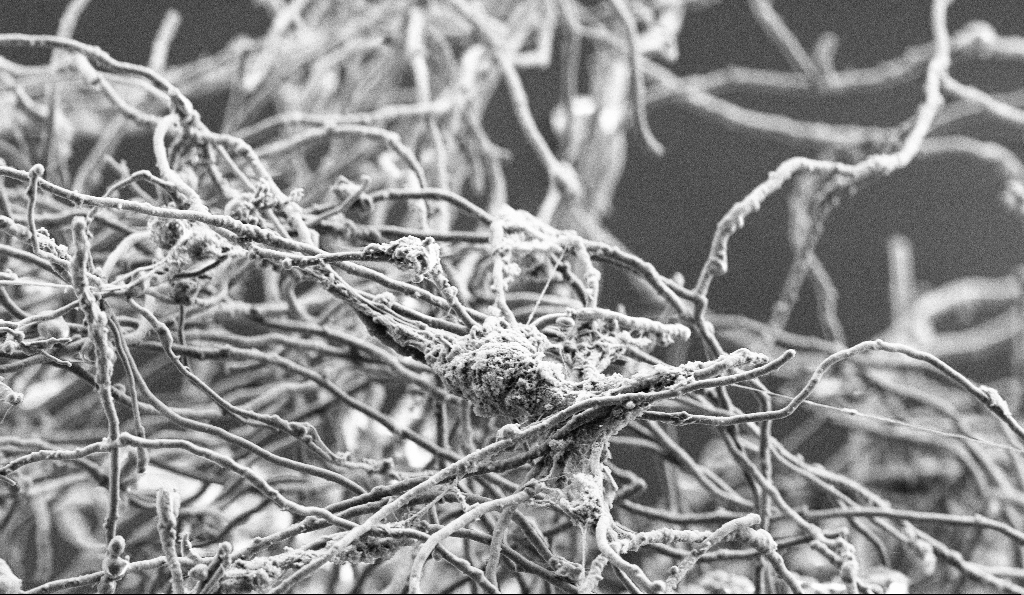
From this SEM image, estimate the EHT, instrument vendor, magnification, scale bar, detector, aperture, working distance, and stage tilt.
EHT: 3 kV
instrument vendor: Zeiss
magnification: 5 K X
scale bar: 10000 nm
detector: SE2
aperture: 30 µm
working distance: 4.9 mm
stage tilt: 0°